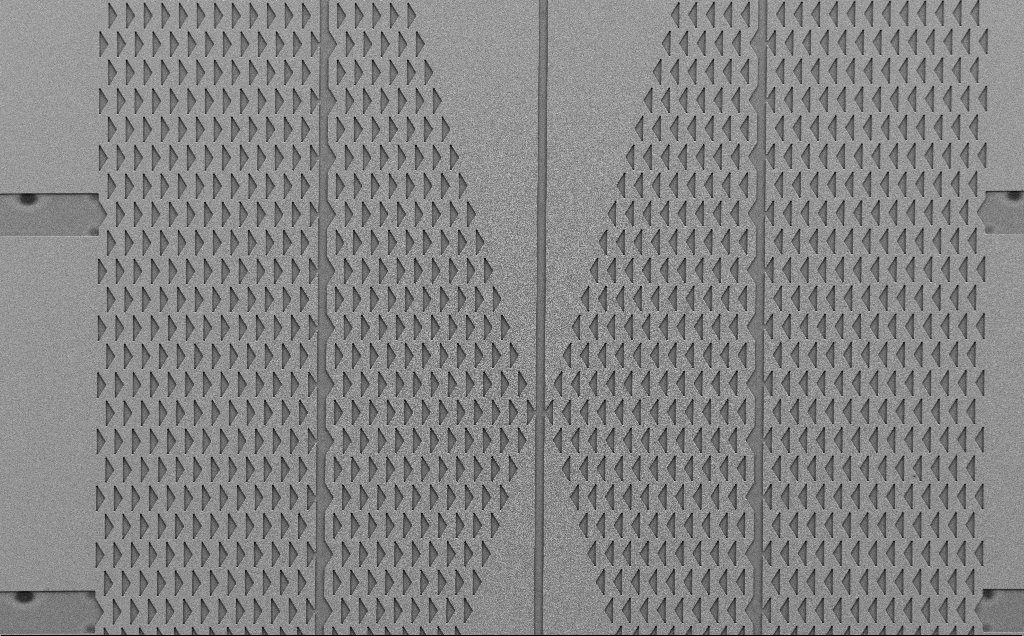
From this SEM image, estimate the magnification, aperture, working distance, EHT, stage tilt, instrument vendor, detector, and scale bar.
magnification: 0.27 K X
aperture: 30 µm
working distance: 6 mm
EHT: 5 kV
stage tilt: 0°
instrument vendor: Zeiss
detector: SE2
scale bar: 100000 nm